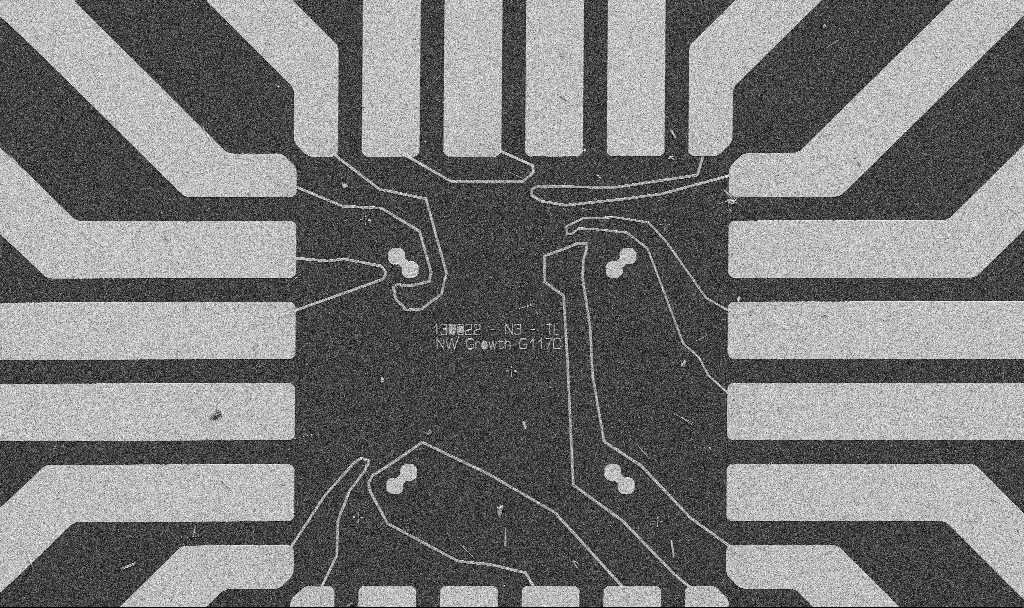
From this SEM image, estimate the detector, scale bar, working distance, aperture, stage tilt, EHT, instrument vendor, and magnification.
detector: SE2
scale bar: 20000 nm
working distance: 8.7 mm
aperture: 30 µm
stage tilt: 0°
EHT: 5 kV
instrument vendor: Zeiss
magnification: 1 K X